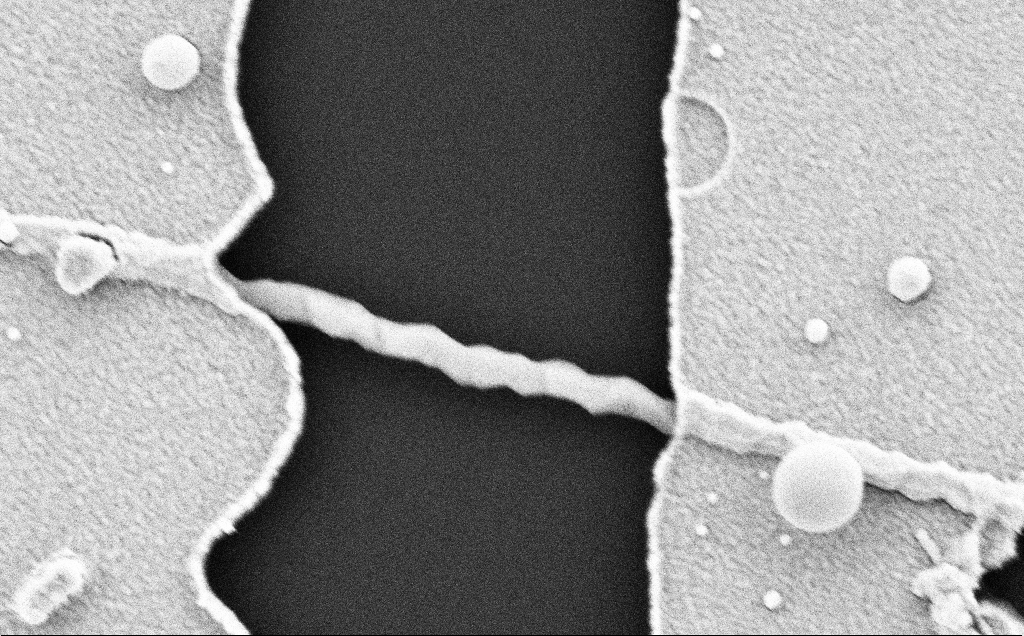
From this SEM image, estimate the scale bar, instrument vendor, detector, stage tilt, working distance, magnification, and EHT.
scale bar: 200 nm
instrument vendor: Zeiss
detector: SE2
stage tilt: -0.7°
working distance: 8 mm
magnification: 76.46 K X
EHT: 5 kV